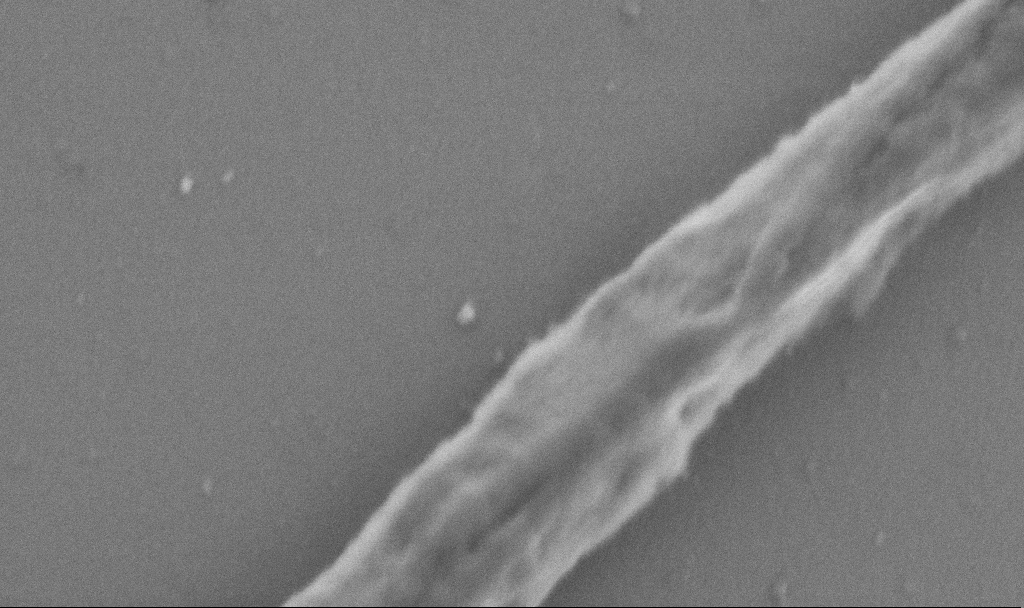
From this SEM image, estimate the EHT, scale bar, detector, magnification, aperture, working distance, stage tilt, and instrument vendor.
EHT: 1 kV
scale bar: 200 nm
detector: SE2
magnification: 80 K X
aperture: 30 µm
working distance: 6.9 mm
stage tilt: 0°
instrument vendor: Zeiss